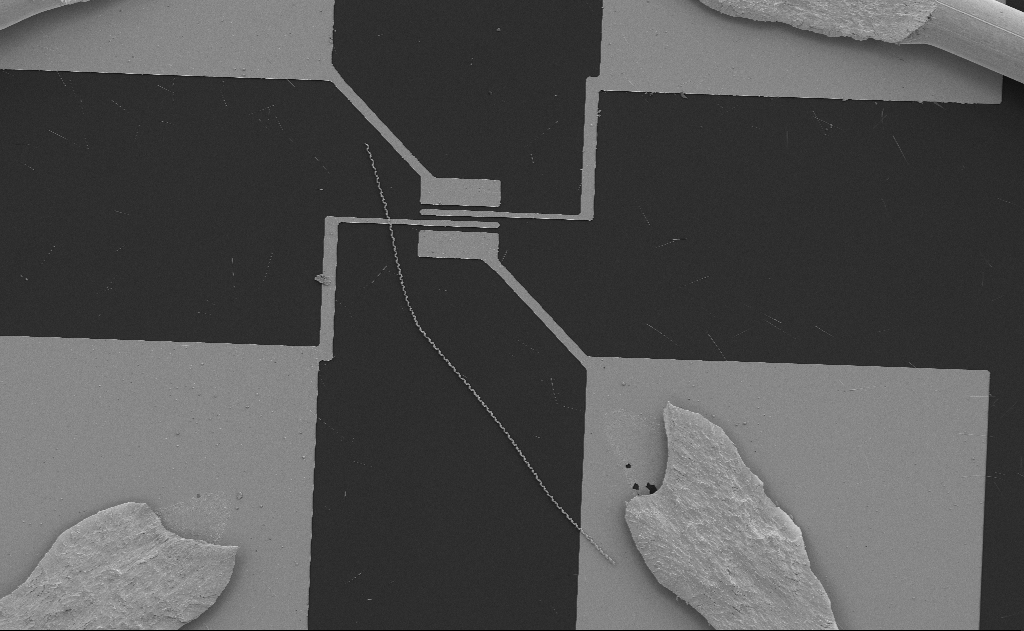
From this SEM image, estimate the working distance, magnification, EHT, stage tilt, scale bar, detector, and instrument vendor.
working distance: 13 mm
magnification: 0.986 K X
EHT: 5 kV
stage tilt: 0°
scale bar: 20000 nm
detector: SE2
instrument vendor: Zeiss